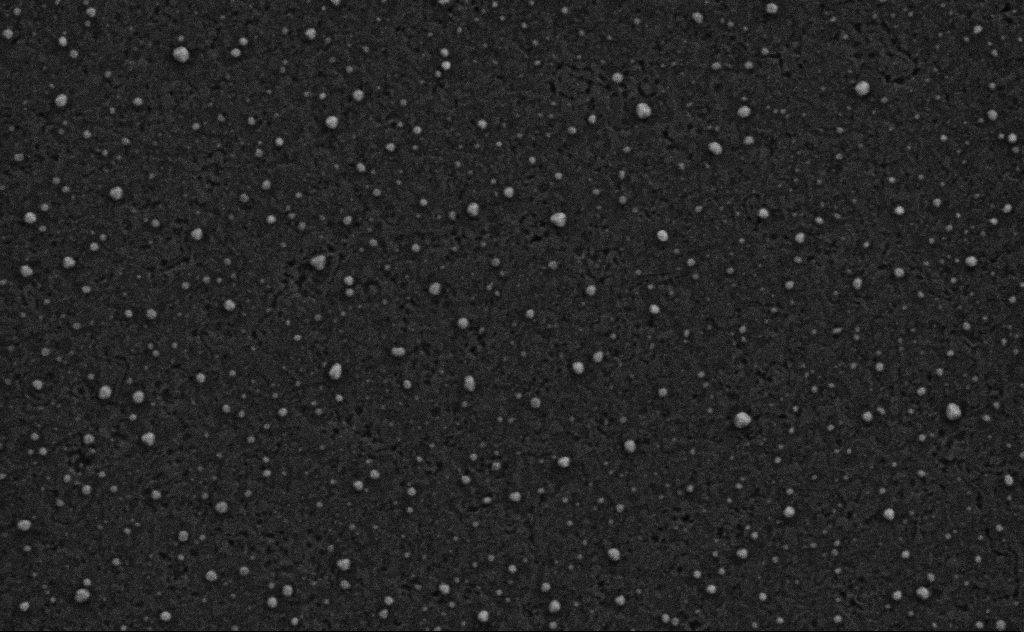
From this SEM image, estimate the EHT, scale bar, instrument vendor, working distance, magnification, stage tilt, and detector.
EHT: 3 kV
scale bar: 200 nm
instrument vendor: Zeiss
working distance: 4 mm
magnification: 80 K X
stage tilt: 0°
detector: SE2